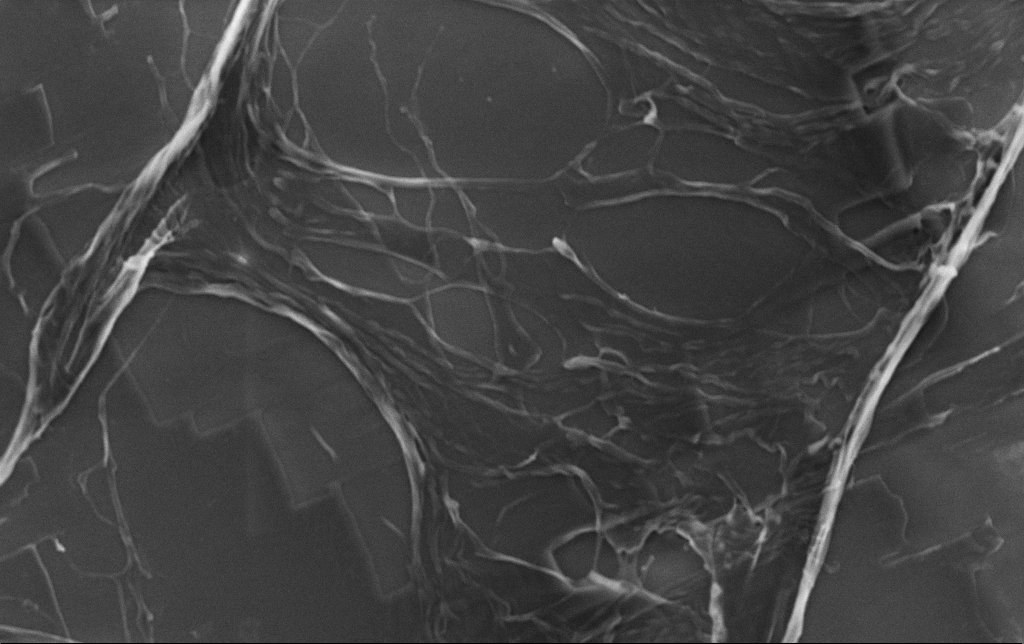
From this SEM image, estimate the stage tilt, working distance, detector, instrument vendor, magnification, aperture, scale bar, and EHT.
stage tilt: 0°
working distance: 3.1 mm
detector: InLens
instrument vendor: Zeiss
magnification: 13.17 K X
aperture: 30 µm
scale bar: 2000 nm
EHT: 5 kV